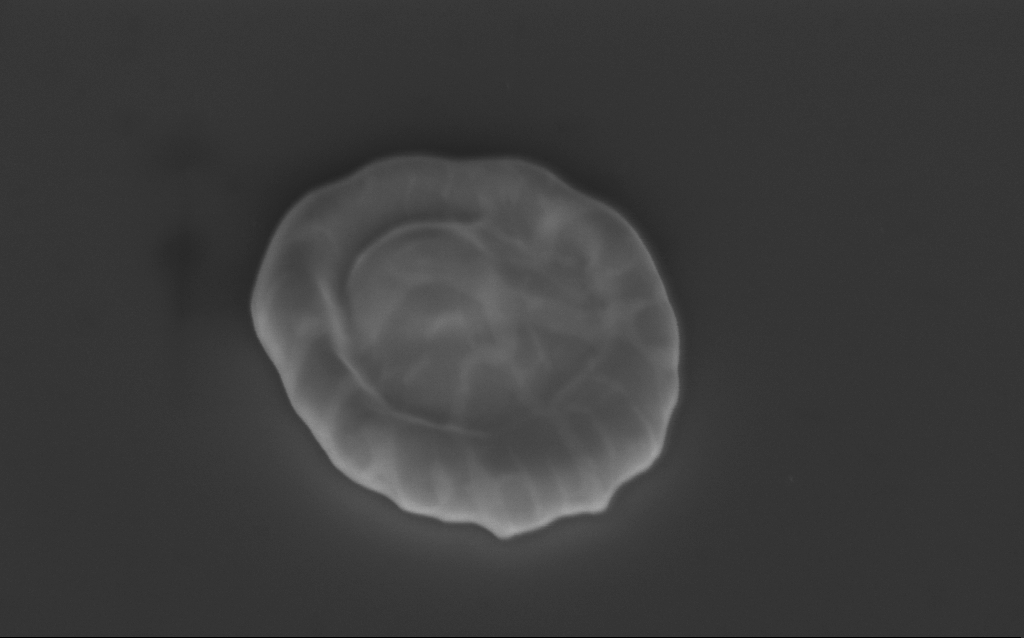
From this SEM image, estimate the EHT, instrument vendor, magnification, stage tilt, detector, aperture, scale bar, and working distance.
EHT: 3 kV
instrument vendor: Zeiss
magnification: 78.62 K X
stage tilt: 40°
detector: InLens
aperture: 30 µm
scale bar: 200 nm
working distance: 5 mm